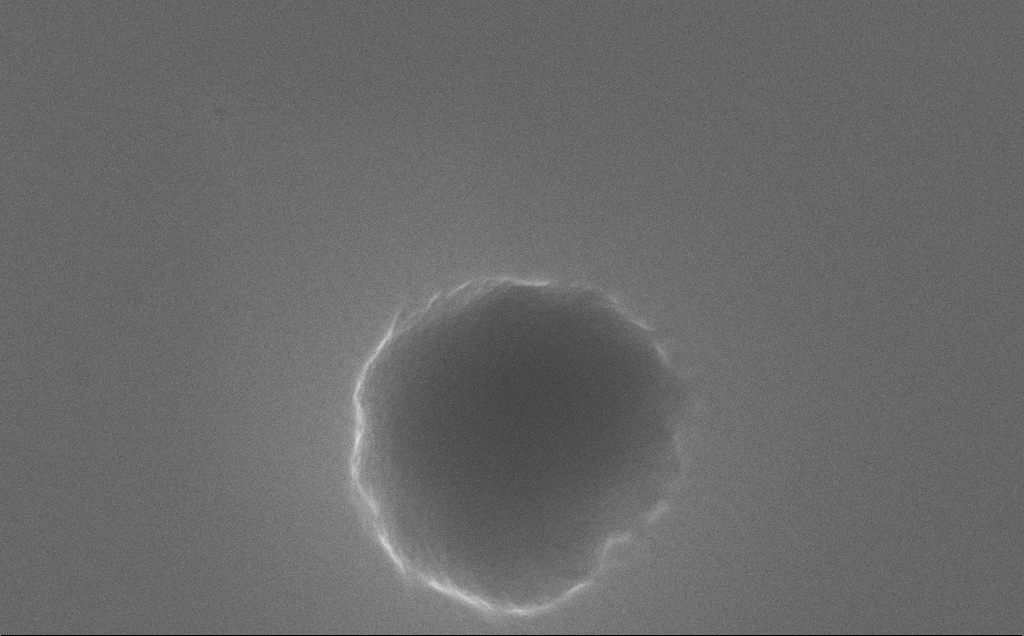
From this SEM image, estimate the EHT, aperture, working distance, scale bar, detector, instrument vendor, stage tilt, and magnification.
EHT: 10 kV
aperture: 30 µm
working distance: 16 mm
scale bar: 1000 nm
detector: SE2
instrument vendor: Zeiss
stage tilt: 0°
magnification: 63.52 K X